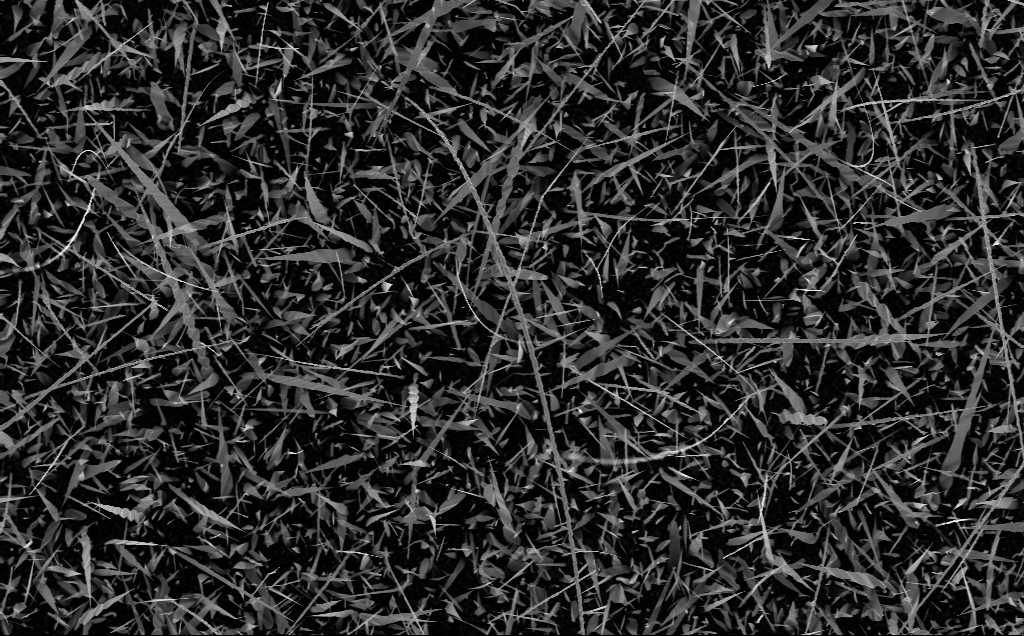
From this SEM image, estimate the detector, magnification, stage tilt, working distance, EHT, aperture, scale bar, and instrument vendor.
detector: InLens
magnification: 5 K X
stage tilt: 0°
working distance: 6 mm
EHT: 10 kV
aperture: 30 µm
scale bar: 10000 nm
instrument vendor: Zeiss